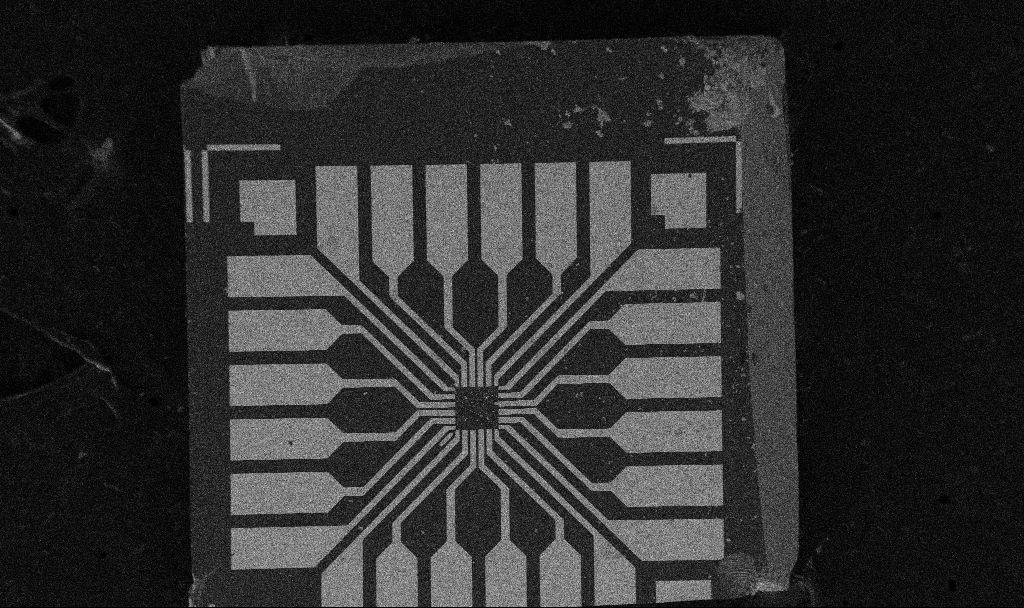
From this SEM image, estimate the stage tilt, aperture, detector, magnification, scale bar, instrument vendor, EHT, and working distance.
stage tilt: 0°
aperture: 30 µm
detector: SE2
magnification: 0.1 K X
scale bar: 200000 nm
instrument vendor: Zeiss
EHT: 5 kV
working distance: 10.7 mm